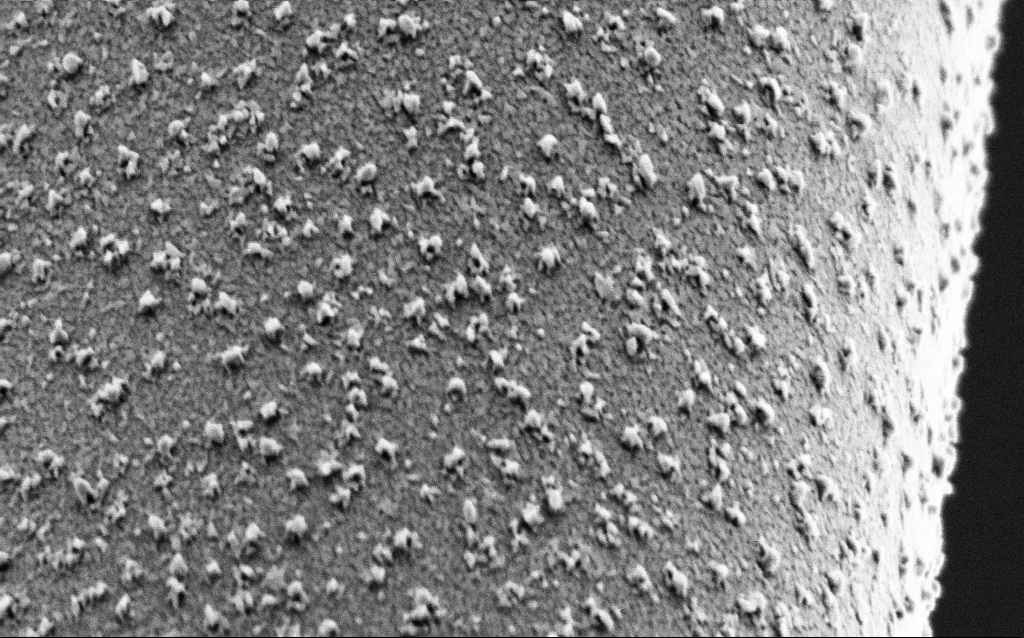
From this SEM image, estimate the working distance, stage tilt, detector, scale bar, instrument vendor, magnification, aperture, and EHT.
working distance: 6.4 mm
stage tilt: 44.9°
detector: SE2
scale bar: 1000 nm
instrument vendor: Zeiss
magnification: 70 K X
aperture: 30 µm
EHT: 2 kV